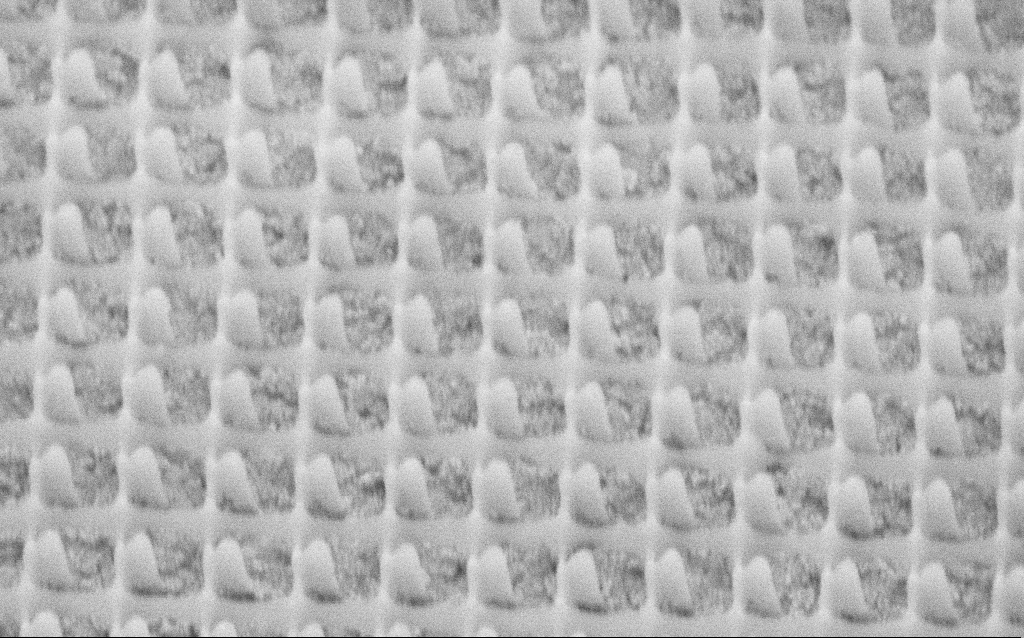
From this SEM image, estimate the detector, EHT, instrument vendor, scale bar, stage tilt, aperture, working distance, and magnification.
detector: SE2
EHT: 3 kV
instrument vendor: Zeiss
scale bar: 1000 nm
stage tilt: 45°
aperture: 30 µm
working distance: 9.7 mm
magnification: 69.79 K X